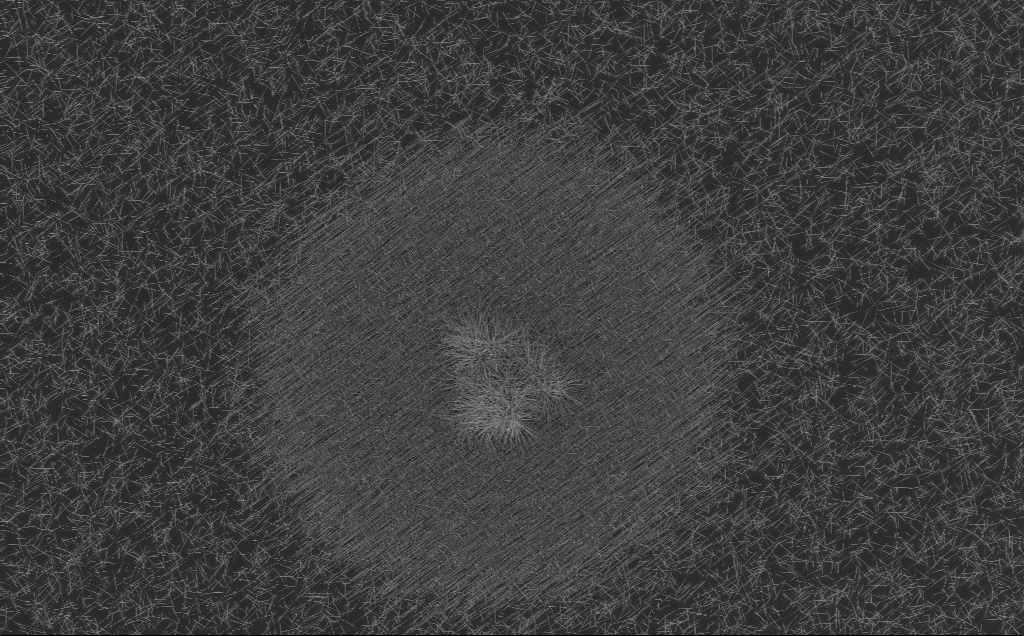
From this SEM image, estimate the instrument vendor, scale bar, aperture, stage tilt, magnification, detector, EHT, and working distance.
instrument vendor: Zeiss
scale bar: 20000 nm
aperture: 30 µm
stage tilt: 0°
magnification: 2.31 K X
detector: InLens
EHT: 10 kV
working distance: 6 mm